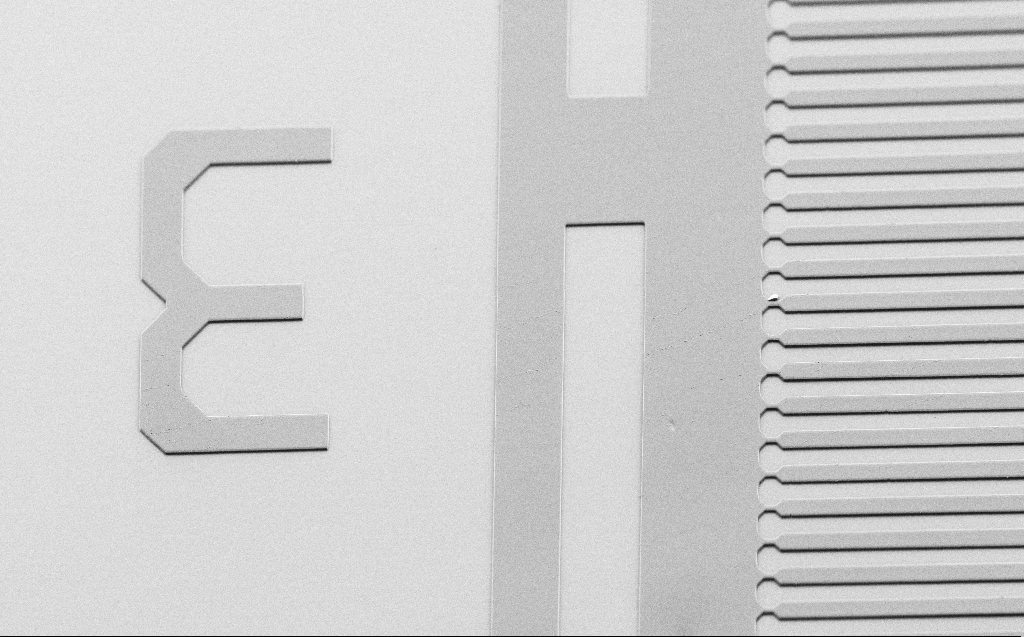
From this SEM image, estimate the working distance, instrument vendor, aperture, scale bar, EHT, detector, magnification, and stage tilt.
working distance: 6 mm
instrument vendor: Zeiss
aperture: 30 µm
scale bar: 100000 nm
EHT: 1.7 kV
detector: SE2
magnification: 0.5 K X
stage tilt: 45°